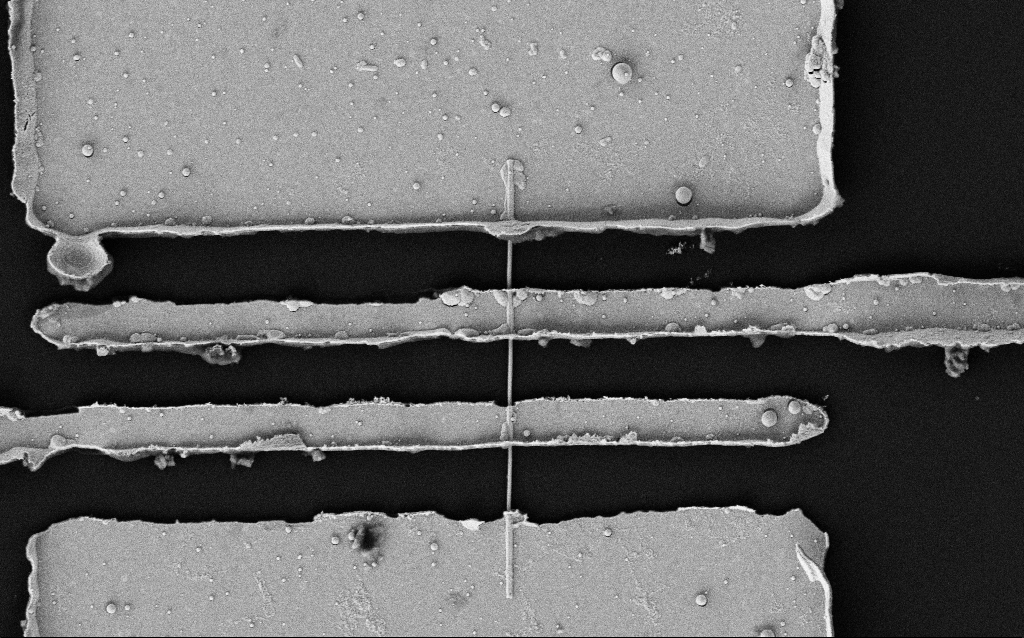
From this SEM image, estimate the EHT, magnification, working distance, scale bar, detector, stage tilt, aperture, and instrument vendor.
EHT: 5 kV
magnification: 9.84 K X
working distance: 9.3 mm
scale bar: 2000 nm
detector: SE2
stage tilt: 0°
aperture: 30 µm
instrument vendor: Zeiss